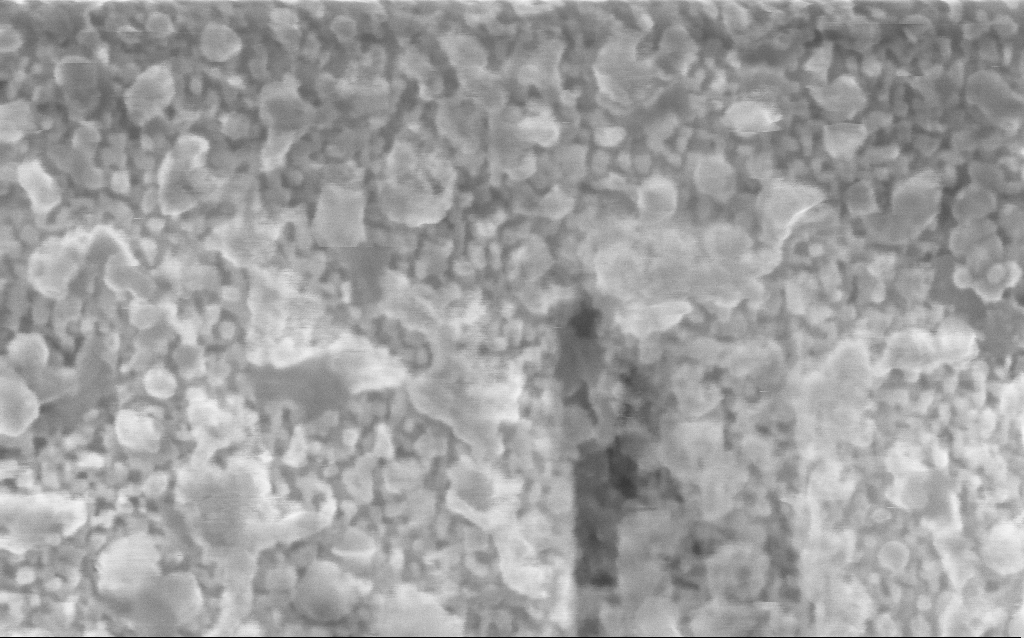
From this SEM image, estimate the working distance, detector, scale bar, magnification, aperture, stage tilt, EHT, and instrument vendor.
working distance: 4.2 mm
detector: InLens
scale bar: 100 nm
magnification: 416.56 K X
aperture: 30 µm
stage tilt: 0°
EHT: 10 kV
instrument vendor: Zeiss